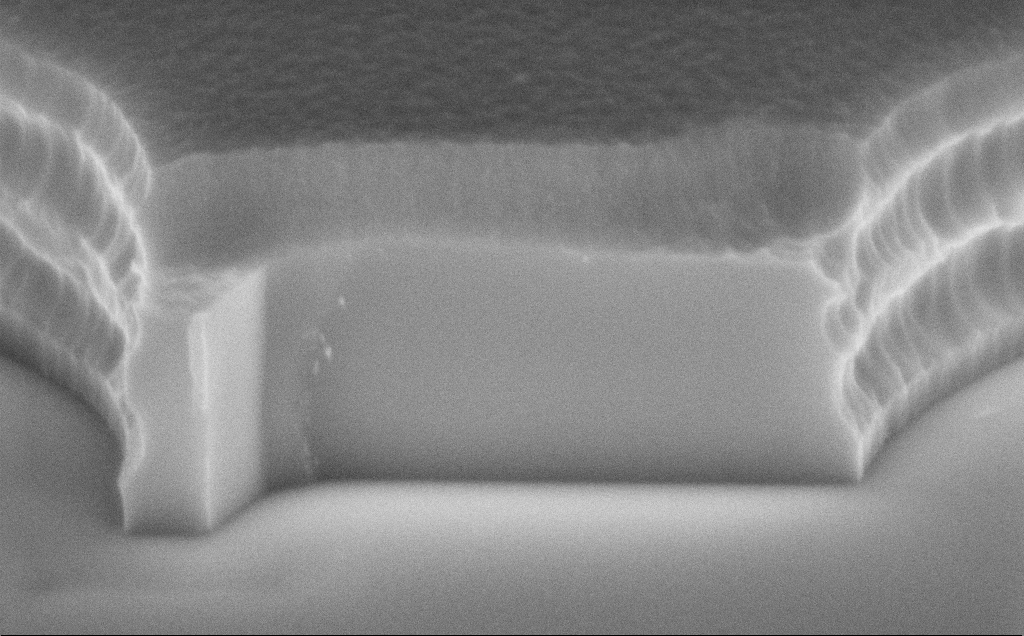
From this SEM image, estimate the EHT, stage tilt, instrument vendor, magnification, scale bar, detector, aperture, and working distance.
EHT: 8 kV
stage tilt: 70°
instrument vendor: Zeiss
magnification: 54.01 K X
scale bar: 1000 nm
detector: InLens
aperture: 30 µm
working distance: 12 mm